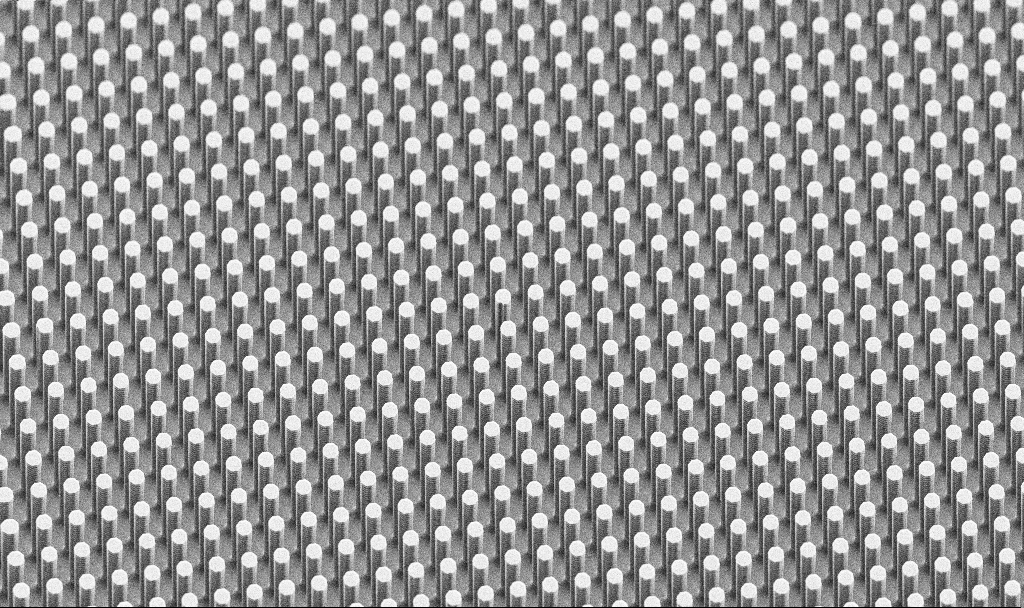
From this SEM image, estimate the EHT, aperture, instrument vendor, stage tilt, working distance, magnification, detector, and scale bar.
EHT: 5 kV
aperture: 30 µm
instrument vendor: Zeiss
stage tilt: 45°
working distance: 7.1 mm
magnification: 2.01 K X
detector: SE2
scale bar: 10000 nm